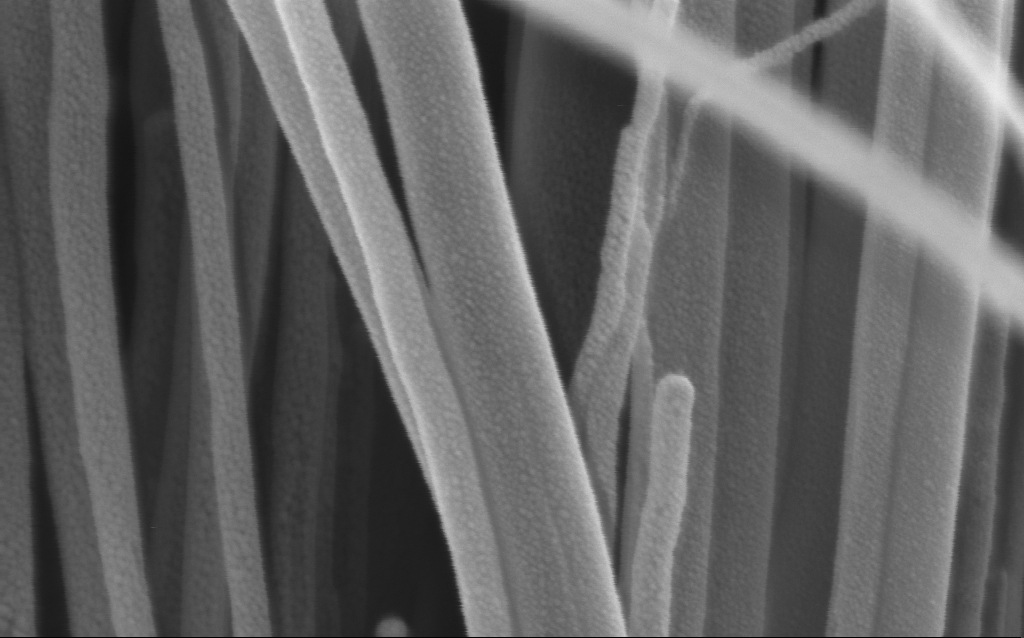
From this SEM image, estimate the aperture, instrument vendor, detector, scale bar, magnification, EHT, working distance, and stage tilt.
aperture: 30 µm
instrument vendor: Zeiss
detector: InLens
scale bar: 200 nm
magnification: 223.79 K X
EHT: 3 kV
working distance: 3 mm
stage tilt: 0°